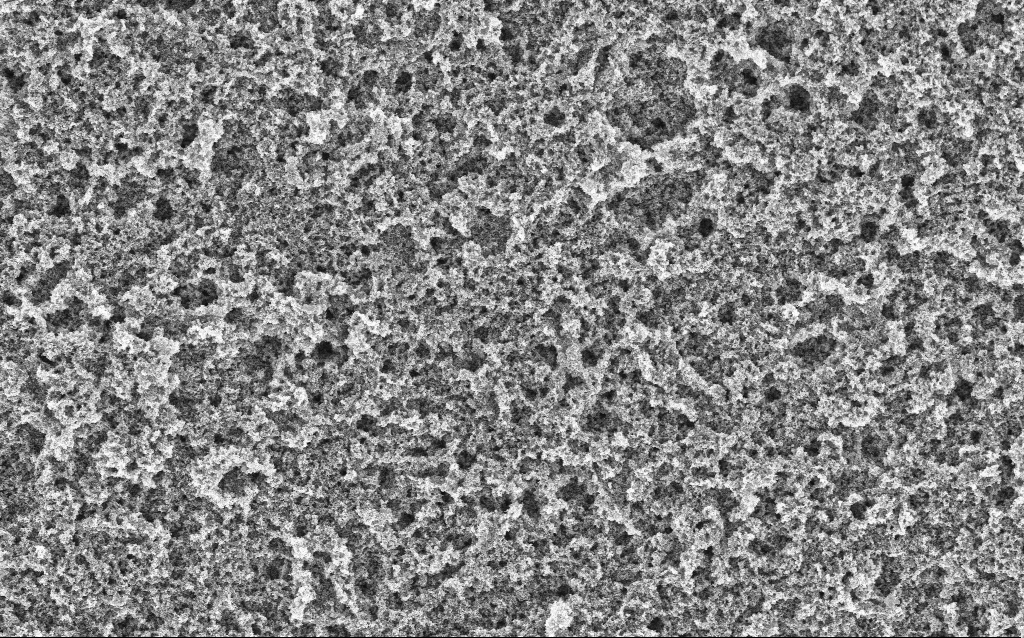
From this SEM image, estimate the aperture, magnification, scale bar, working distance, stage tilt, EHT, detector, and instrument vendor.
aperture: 30 µm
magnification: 15.33 K X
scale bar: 1000 nm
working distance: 4.4 mm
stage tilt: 0°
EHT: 5 kV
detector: InLens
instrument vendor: Zeiss